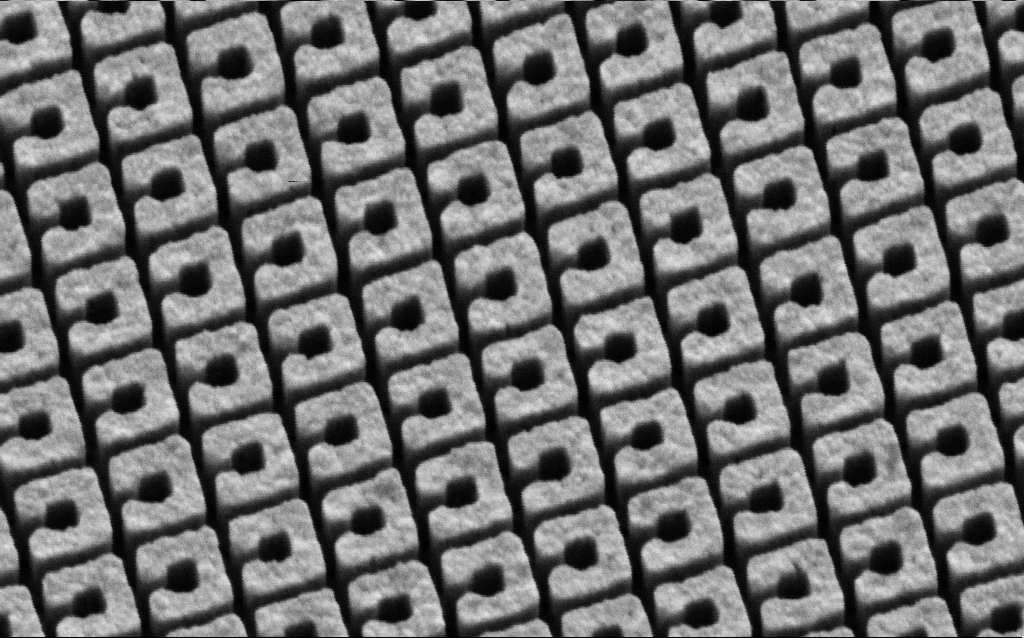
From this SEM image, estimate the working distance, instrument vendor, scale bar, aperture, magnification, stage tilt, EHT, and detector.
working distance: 9.9 mm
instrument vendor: Zeiss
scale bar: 200 nm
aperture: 30 µm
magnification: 76.9 K X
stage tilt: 45°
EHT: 3 kV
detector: SE2